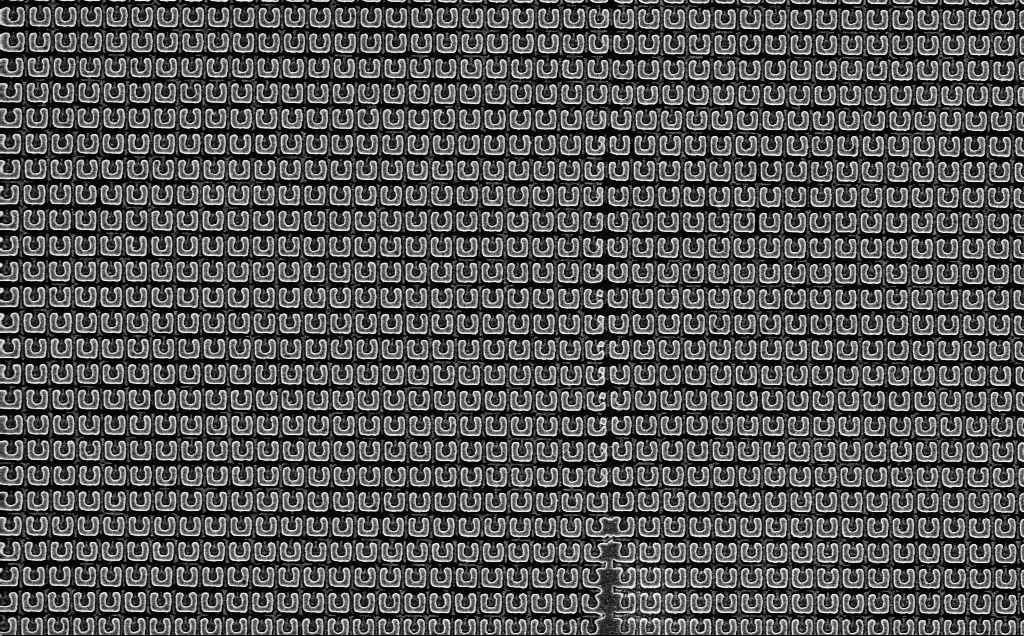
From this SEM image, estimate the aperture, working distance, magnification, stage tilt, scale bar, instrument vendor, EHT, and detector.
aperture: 30 µm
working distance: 2.4 mm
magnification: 20.26 K X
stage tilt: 0°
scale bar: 2000 nm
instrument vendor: Zeiss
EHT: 5 kV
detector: InLens